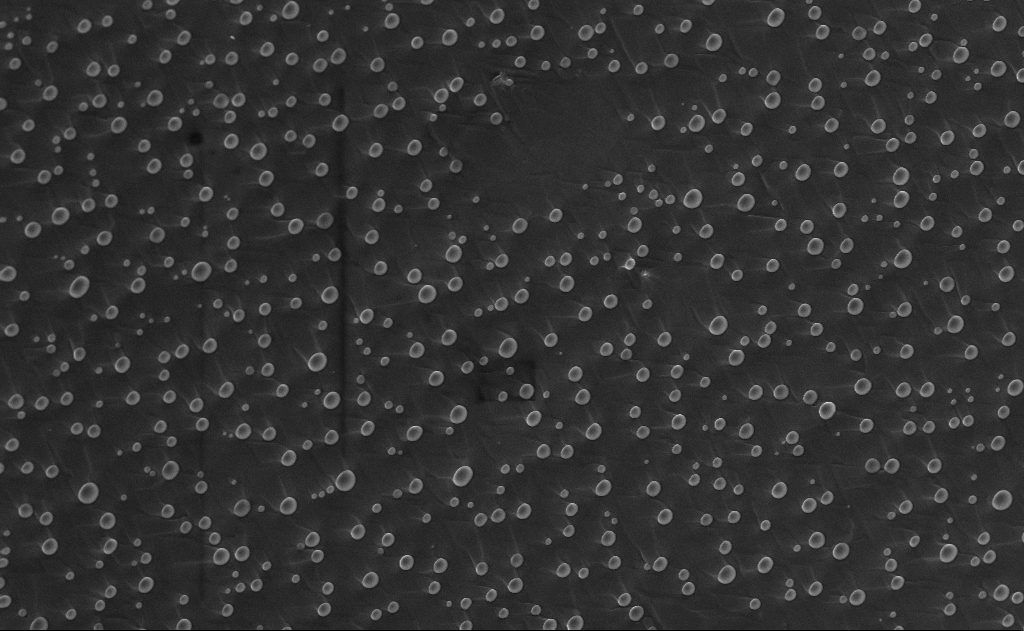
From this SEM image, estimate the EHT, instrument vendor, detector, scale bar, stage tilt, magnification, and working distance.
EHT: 10 kV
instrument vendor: Zeiss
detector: InLens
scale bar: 10000 nm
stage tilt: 0°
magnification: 5 K X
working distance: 11 mm